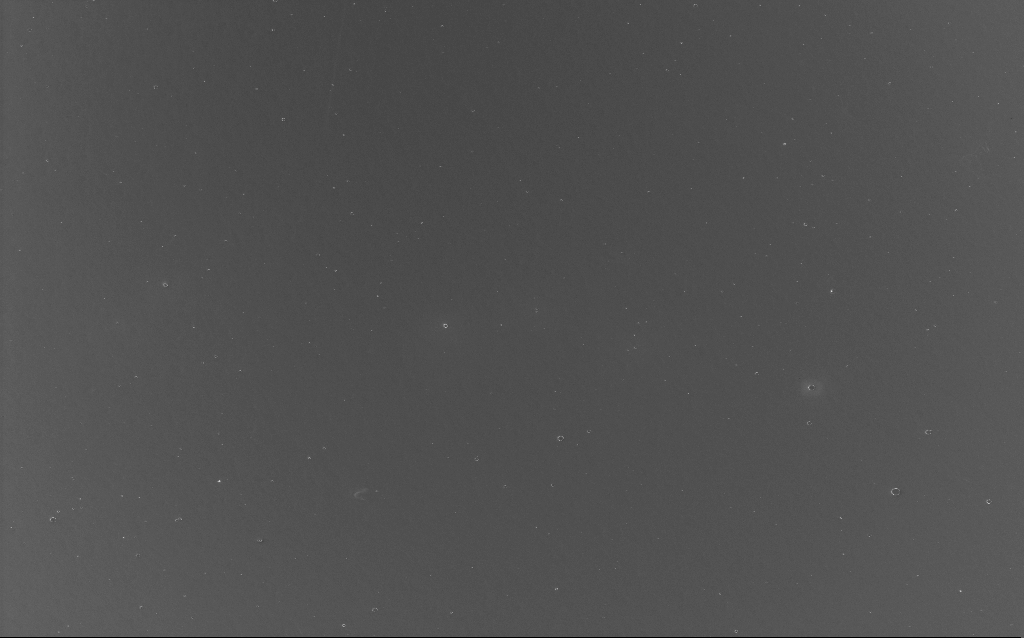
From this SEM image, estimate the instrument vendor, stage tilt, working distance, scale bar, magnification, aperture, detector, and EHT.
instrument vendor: Zeiss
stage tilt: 0°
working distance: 2 mm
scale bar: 20000 nm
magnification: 0.84 K X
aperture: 30 µm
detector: InLens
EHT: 10 kV